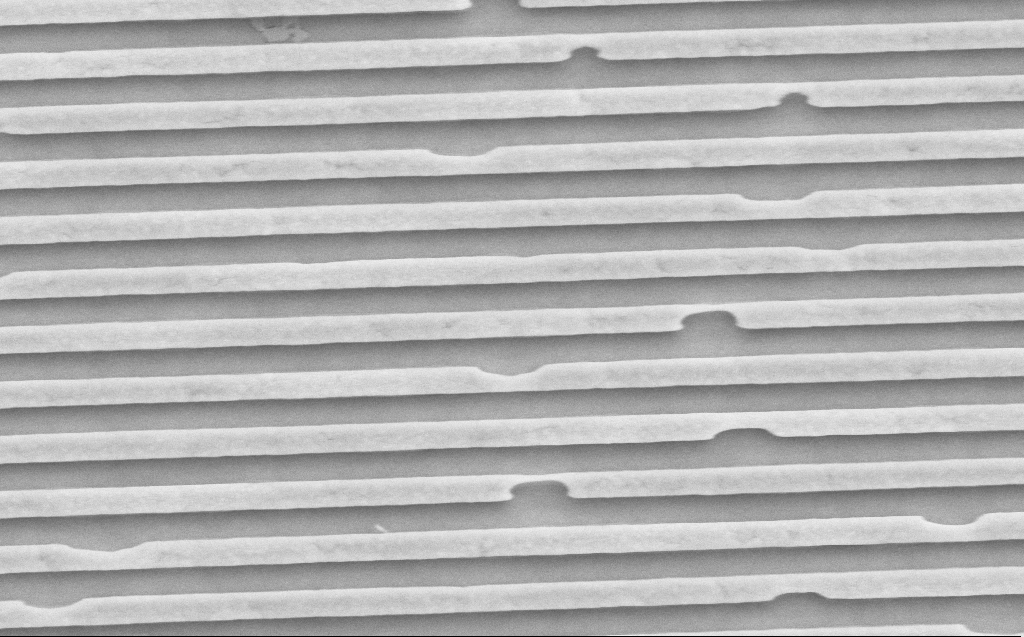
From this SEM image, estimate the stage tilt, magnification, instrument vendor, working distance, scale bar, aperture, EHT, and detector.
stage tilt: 0°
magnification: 39.91 K X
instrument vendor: Zeiss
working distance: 10 mm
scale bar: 1000 nm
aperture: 30 µm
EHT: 10 kV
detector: SE2